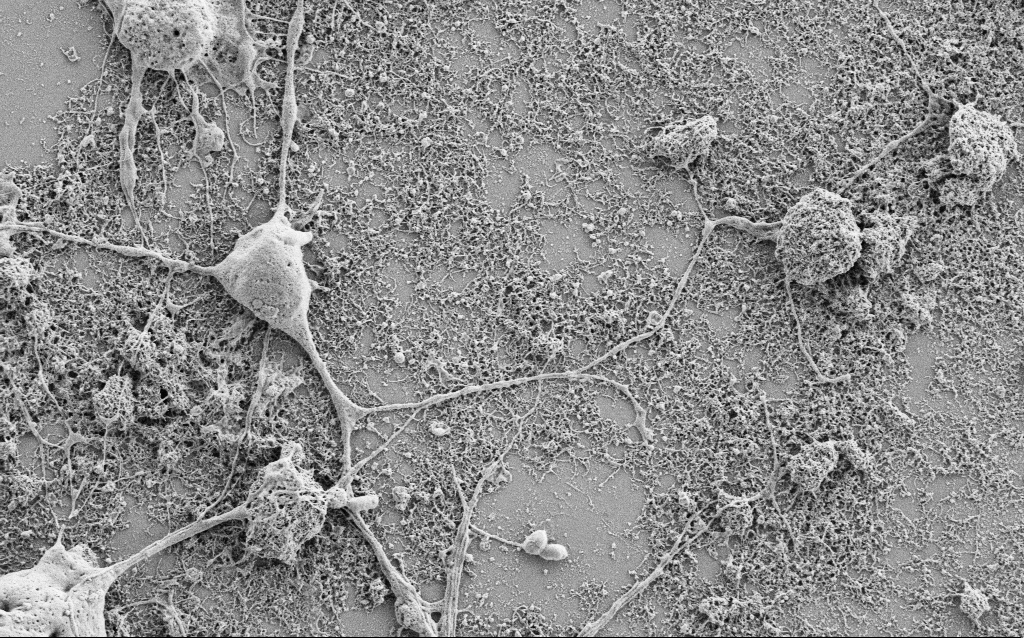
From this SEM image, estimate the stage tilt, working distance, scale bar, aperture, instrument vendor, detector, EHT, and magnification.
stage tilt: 0°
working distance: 6.8 mm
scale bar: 2000 nm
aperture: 30 µm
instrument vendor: Zeiss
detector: SE2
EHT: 1.5 kV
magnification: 10 K X